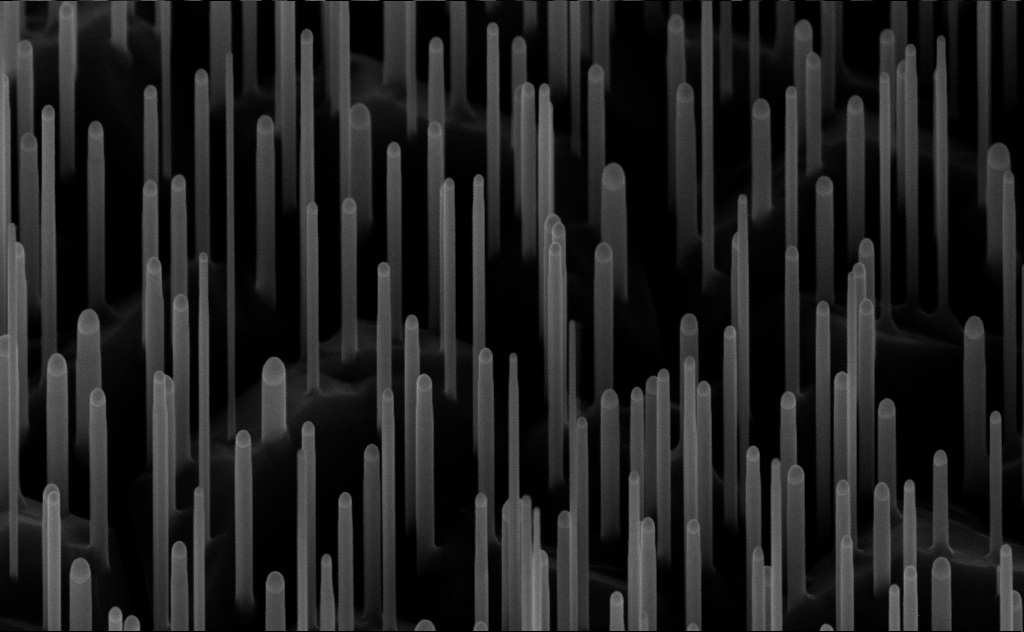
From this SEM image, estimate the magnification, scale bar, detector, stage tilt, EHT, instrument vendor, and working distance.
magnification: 80 K X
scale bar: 200 nm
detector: InLens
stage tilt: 45°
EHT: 10 kV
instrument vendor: Zeiss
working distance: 7 mm